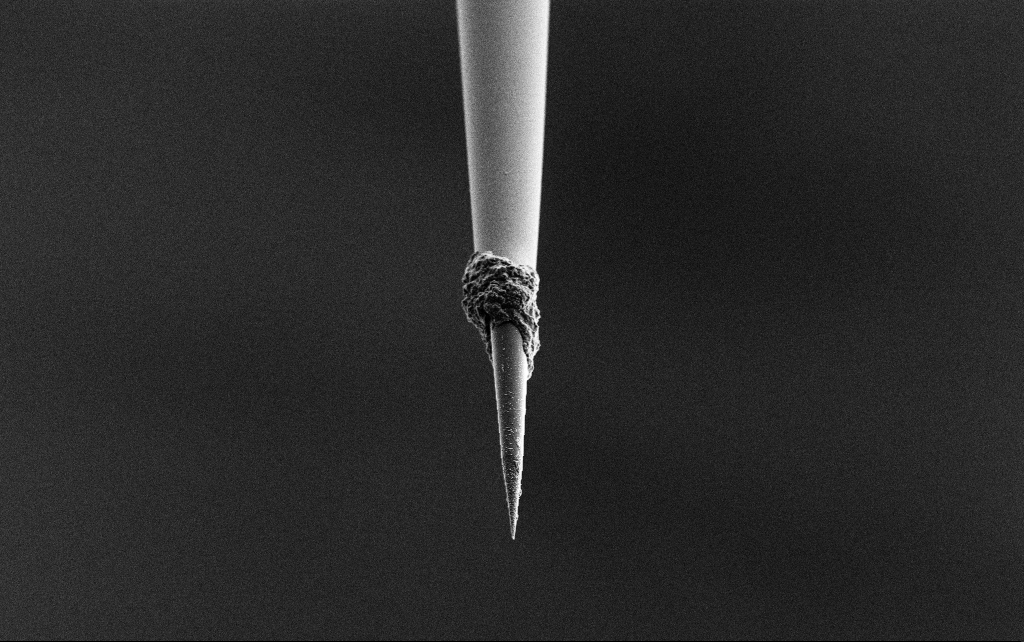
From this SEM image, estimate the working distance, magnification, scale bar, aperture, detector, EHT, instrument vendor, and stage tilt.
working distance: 7.7 mm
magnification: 2.5 K X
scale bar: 20000 nm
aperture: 30 µm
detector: SE2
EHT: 3 kV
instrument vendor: Zeiss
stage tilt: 45°